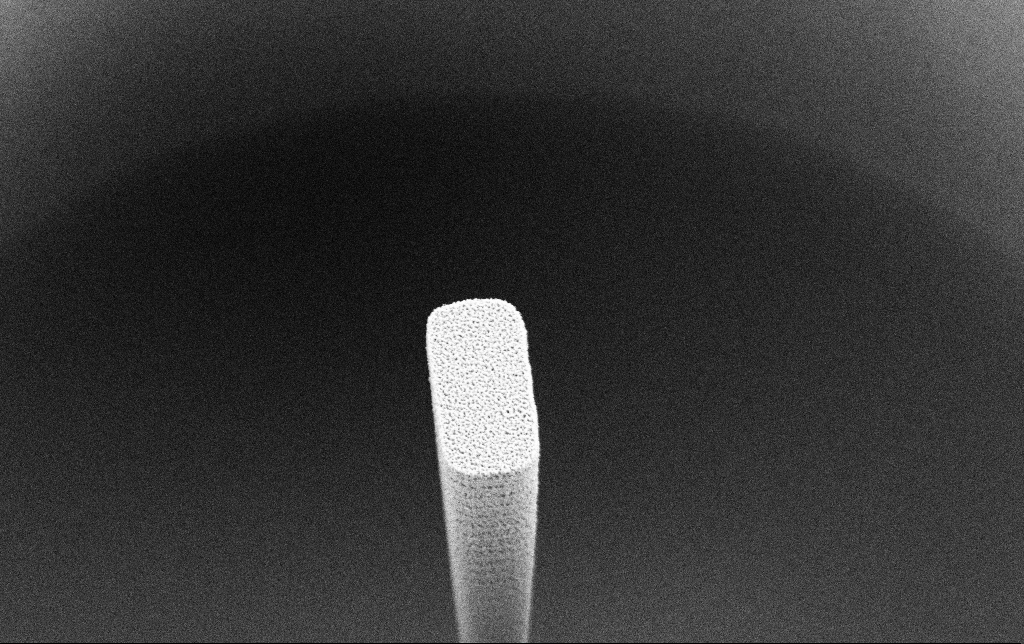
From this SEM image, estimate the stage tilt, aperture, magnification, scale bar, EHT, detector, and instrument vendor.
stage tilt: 27.6°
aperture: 30 µm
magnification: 18.97 K X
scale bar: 2000 nm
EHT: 2 kV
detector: SE2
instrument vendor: Zeiss